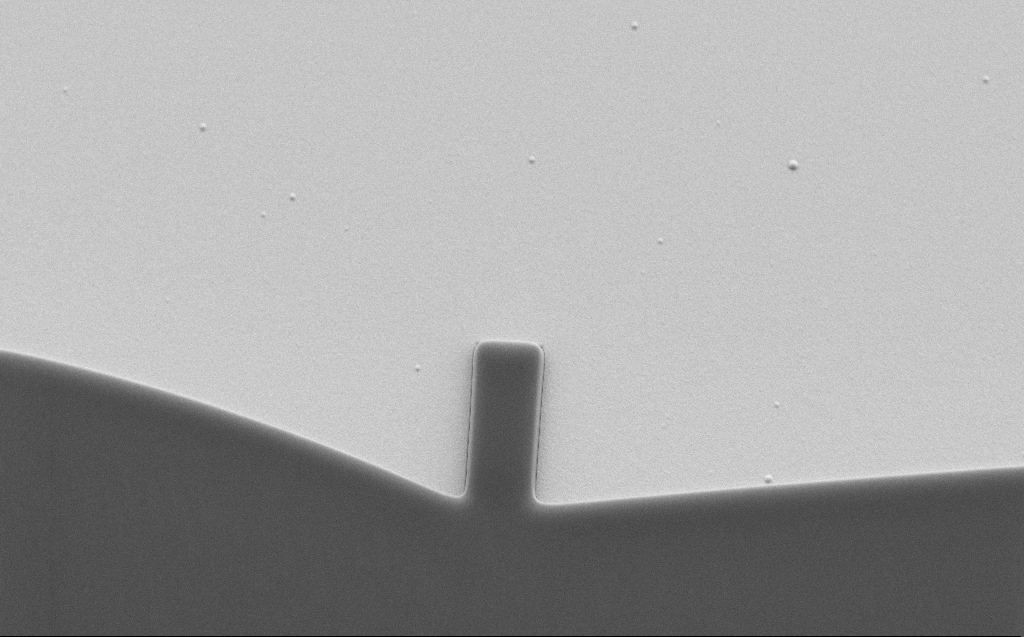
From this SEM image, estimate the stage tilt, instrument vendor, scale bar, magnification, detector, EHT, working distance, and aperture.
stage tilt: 30°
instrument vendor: Zeiss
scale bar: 20000 nm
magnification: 2.87 K X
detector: SE2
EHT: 1.1 kV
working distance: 6 mm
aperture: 30 µm